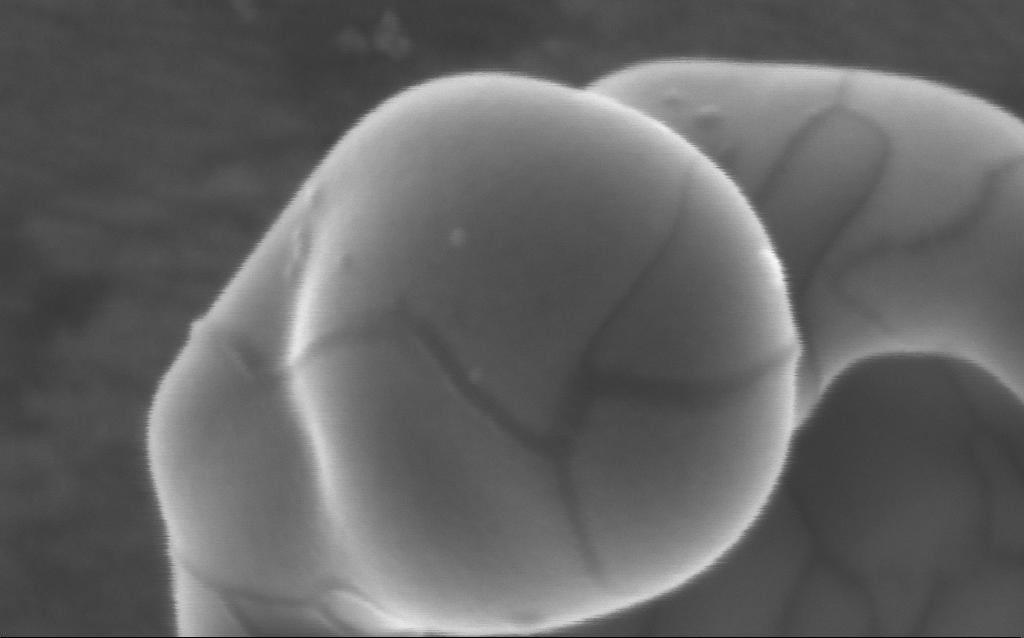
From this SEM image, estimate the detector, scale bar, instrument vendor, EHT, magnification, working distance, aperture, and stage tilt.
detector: InLens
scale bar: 100 nm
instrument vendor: Zeiss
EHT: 5 kV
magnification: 511.26 K X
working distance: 4 mm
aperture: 30 µm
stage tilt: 0°